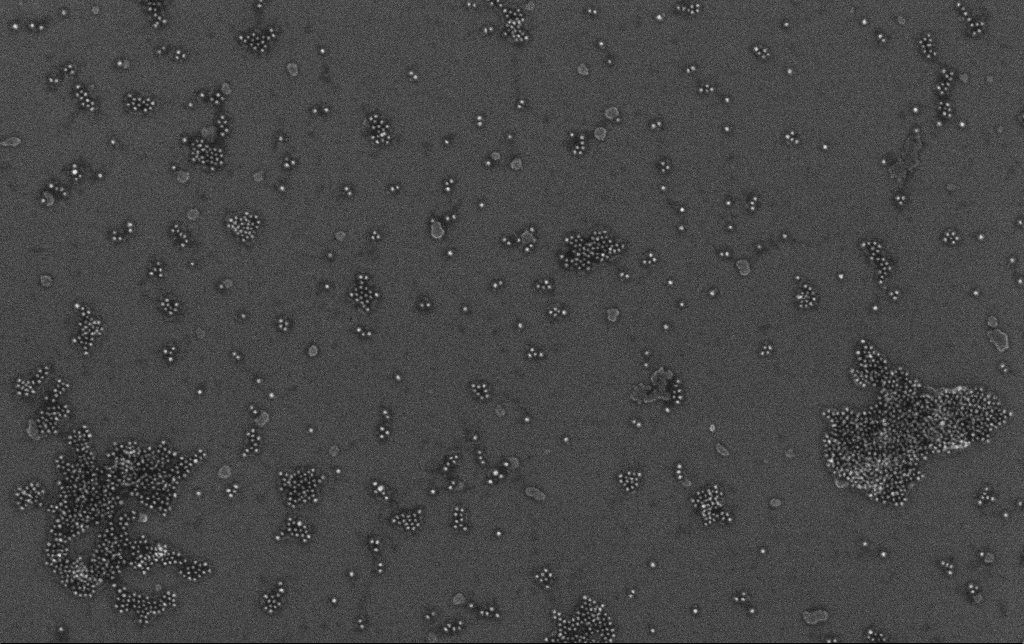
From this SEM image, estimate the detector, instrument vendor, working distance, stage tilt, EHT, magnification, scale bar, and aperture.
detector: InLens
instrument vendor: Zeiss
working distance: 3.4 mm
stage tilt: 0°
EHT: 10 kV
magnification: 100 K X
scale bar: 200 nm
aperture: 30 µm